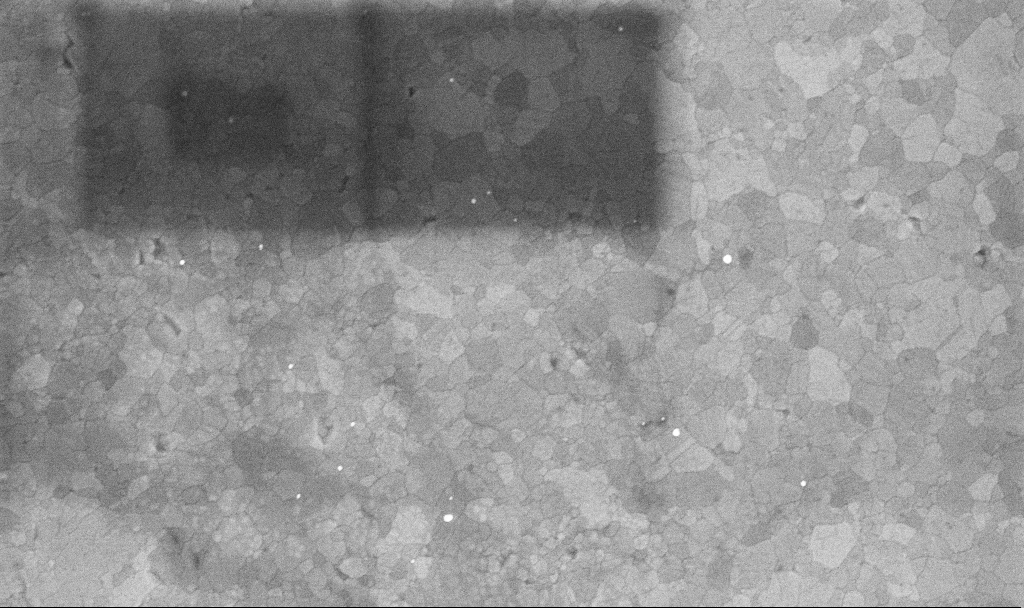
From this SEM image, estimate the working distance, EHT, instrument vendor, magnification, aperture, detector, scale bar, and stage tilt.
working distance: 3.4 mm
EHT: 10 kV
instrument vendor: Zeiss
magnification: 50.95 K X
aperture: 30 µm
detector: InLens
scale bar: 1000 nm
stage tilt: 0°